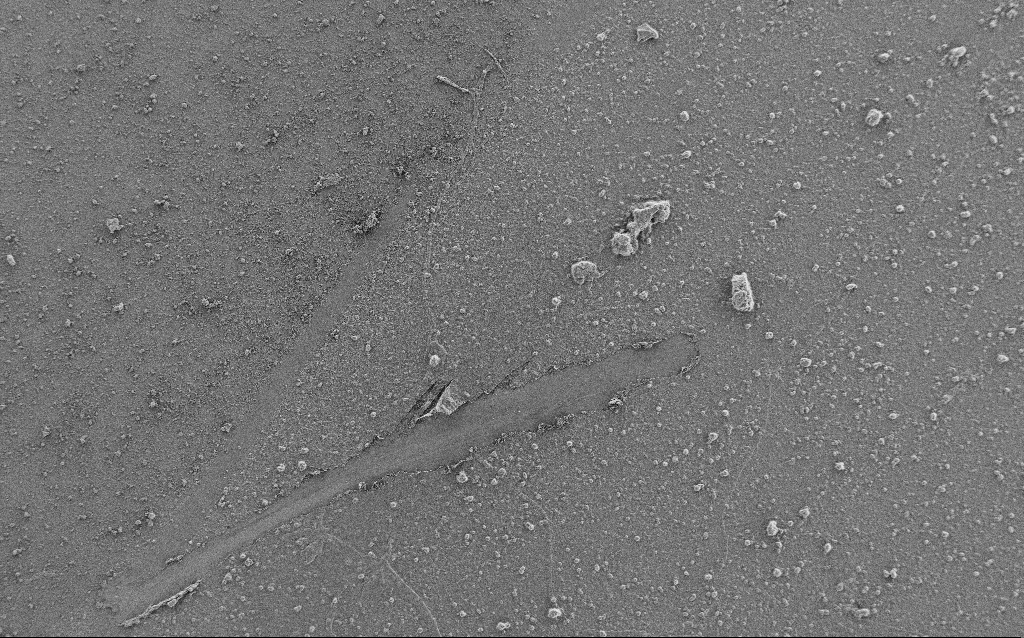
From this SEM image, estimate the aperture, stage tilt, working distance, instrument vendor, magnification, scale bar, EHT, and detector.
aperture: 30 µm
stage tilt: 0°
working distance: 4 mm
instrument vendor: Zeiss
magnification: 0.5 K X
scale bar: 100000 nm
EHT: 1 kV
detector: SE2